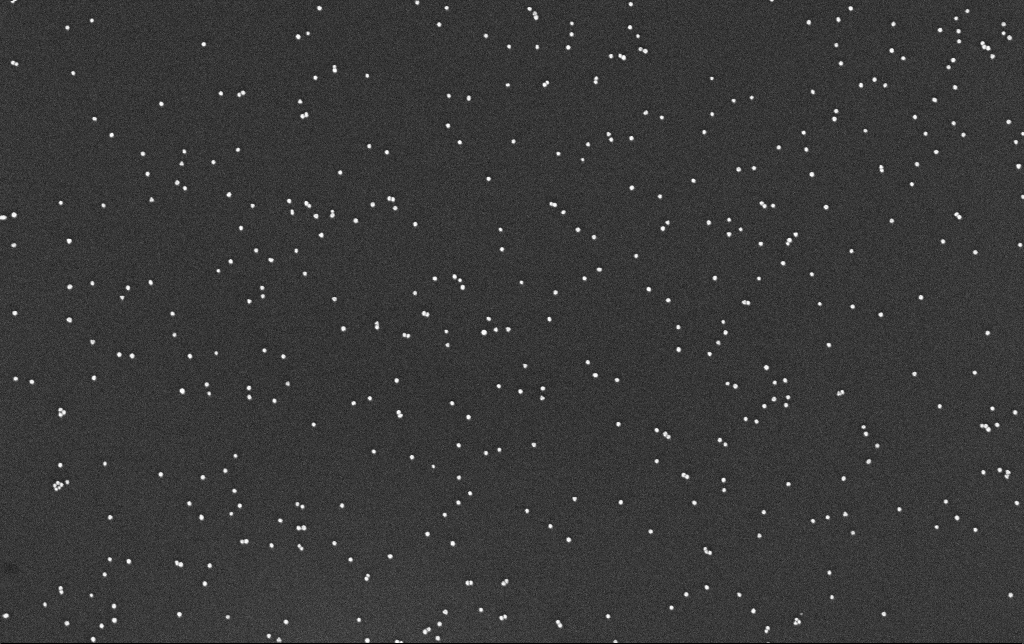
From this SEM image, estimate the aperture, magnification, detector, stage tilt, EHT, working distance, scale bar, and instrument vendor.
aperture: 30 µm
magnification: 100 K X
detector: InLens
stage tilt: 0°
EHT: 10 kV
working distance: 3.4 mm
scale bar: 200 nm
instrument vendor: Zeiss